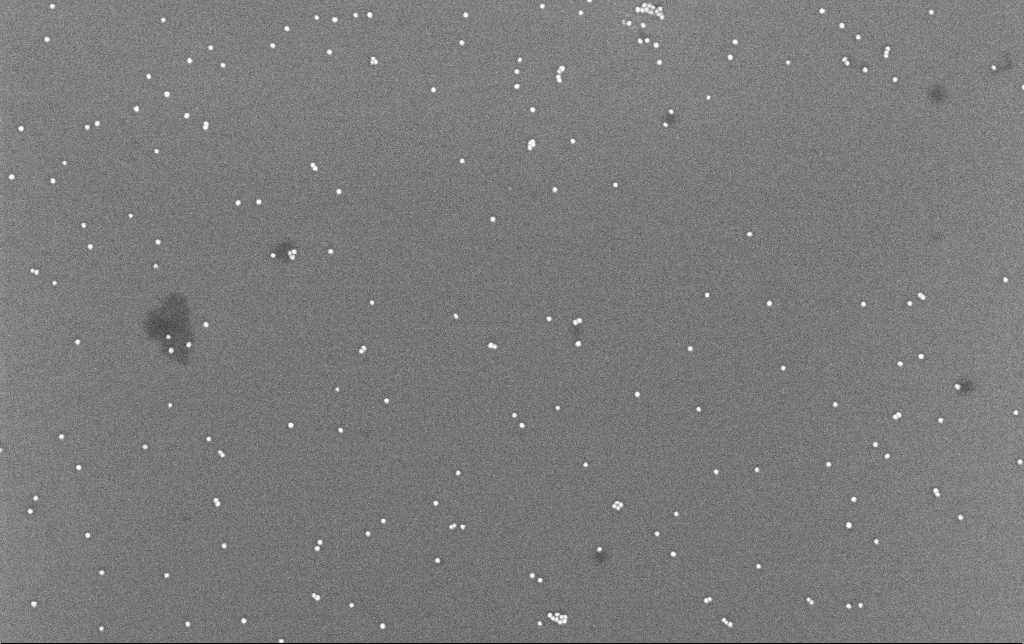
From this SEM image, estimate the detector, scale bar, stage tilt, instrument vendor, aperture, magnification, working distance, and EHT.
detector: InLens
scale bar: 200 nm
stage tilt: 0°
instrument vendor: Zeiss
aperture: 30 µm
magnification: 100 K X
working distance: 6.6 mm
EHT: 8 kV